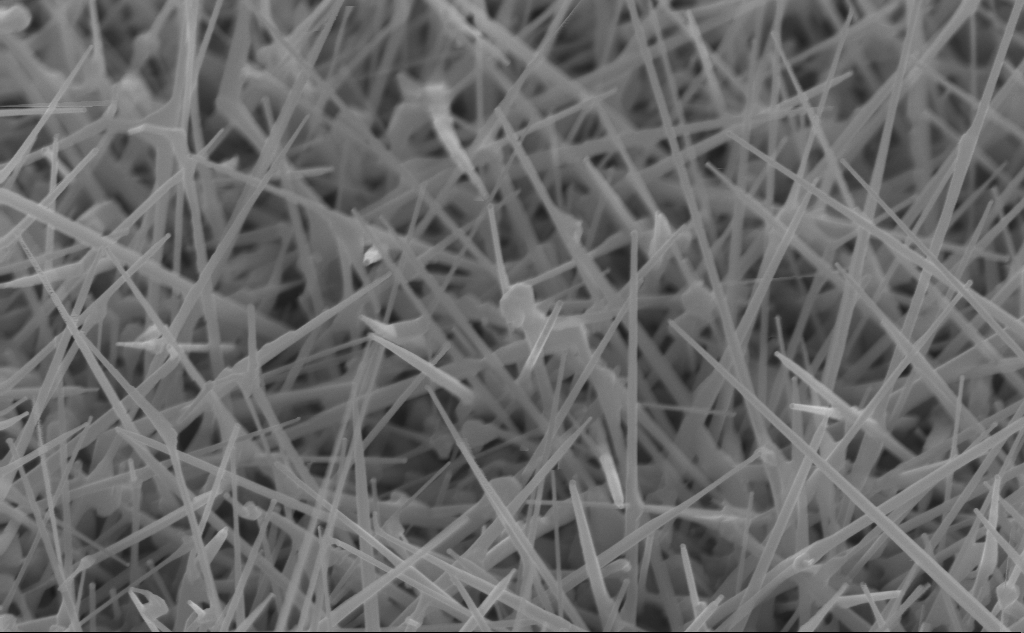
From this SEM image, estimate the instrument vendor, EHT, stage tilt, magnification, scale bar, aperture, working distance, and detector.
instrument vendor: Zeiss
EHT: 10 kV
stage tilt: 45°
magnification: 56.96 K X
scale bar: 1000 nm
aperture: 30 µm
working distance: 4 mm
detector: InLens